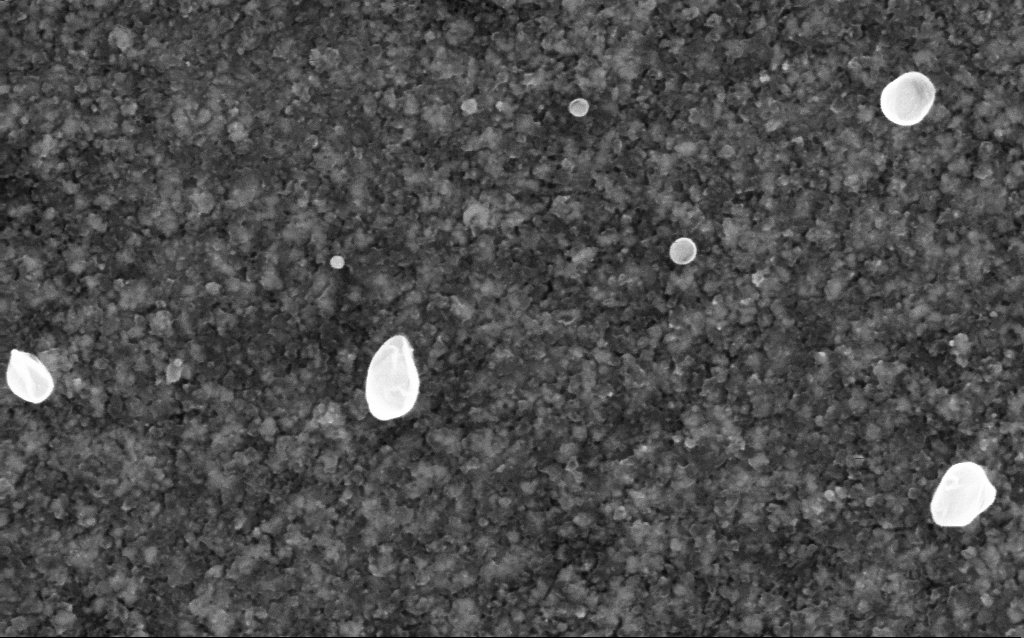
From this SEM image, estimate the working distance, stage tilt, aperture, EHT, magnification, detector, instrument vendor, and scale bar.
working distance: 2.6 mm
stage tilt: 0°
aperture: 30 µm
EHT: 5 kV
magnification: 200 K X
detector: InLens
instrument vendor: Zeiss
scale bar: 100 nm